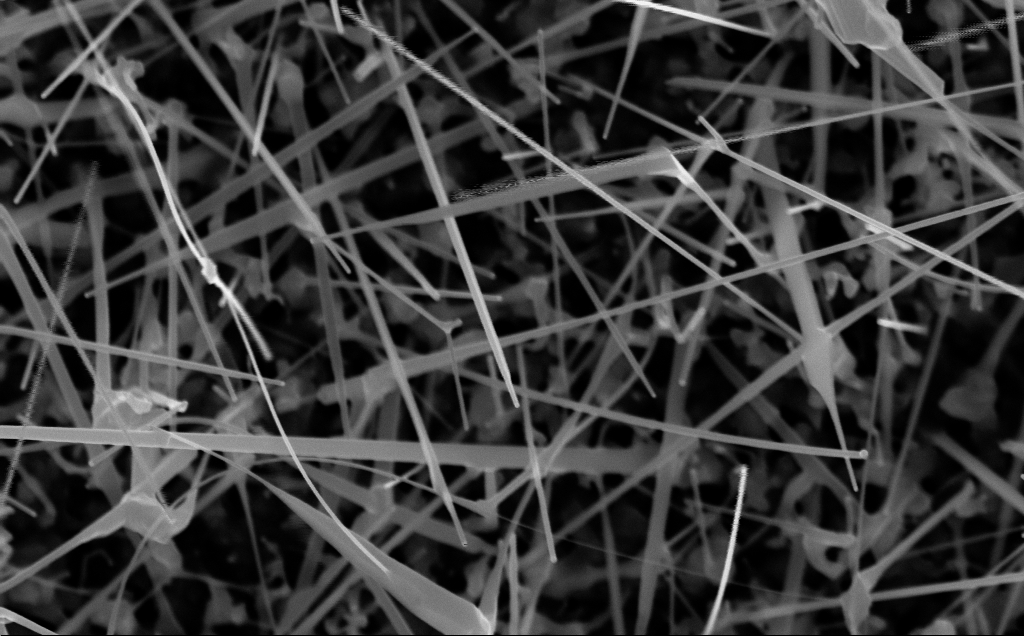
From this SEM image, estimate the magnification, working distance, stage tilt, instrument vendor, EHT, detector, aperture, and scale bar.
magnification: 62.42 K X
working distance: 7 mm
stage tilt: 0°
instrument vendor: Zeiss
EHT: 10 kV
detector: InLens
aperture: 30 µm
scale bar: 1000 nm